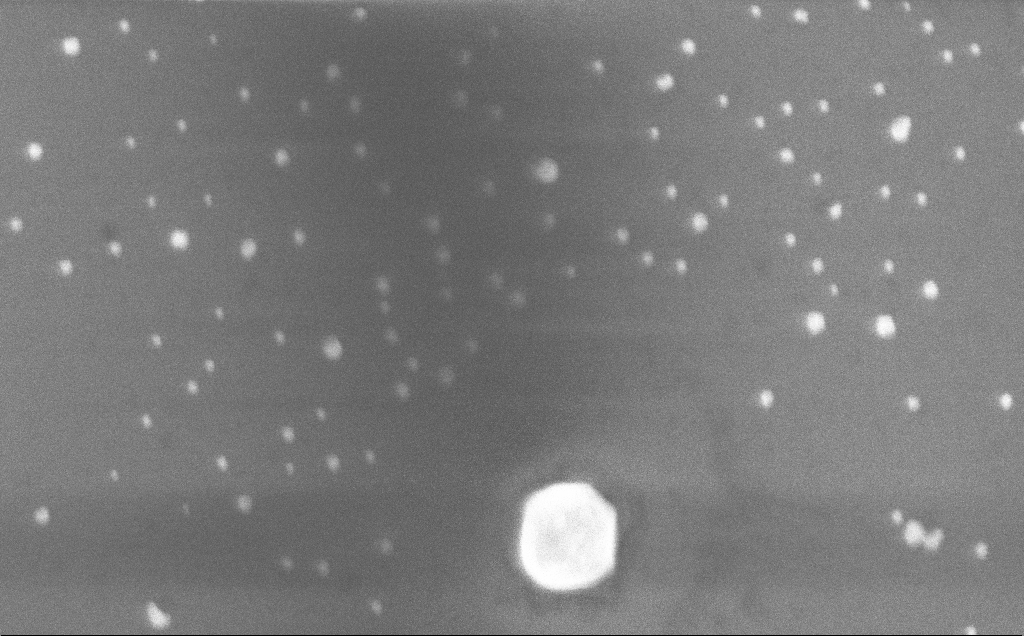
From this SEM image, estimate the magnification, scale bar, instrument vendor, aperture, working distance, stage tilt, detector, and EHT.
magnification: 103.39 K X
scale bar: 200 nm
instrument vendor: Zeiss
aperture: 30 µm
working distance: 4 mm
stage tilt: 0°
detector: InLens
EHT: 10 kV